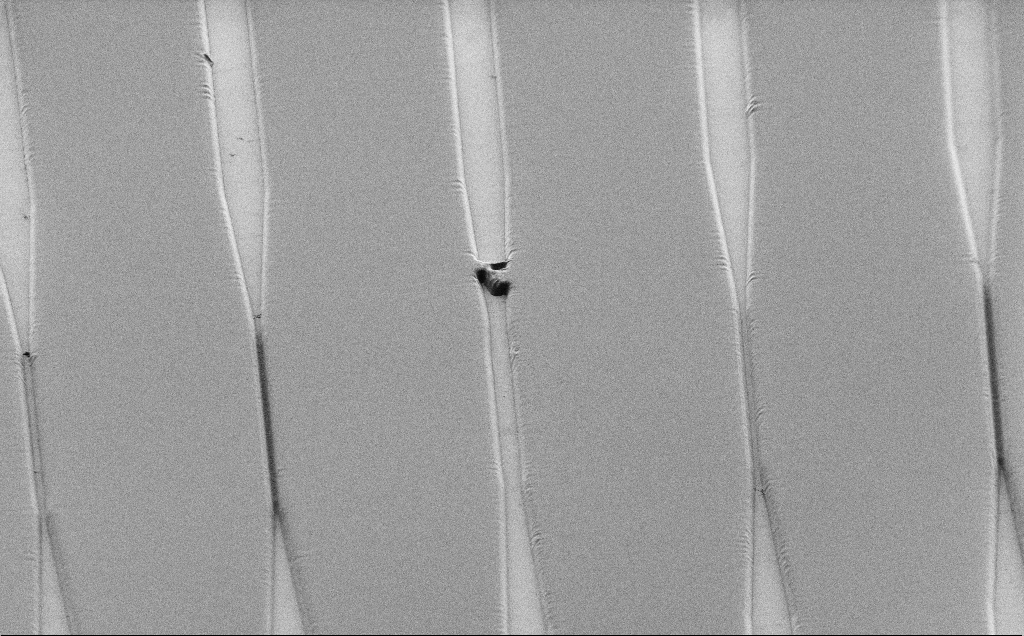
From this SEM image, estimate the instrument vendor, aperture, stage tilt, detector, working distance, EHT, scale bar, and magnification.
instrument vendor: Zeiss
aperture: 30 µm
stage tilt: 45°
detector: SE2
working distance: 8 mm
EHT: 1 kV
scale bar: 20000 nm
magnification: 0.823 K X